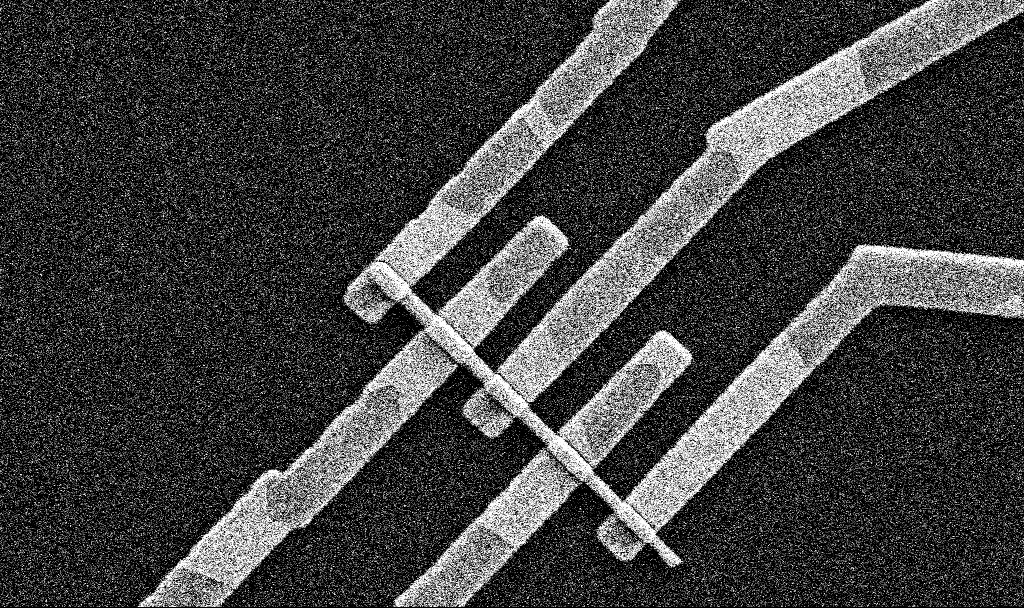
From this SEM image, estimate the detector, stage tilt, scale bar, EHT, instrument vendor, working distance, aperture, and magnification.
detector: SE2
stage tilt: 0°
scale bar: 1000 nm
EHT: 5 kV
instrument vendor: Zeiss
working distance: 8.5 mm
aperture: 30 µm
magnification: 28.5 K X